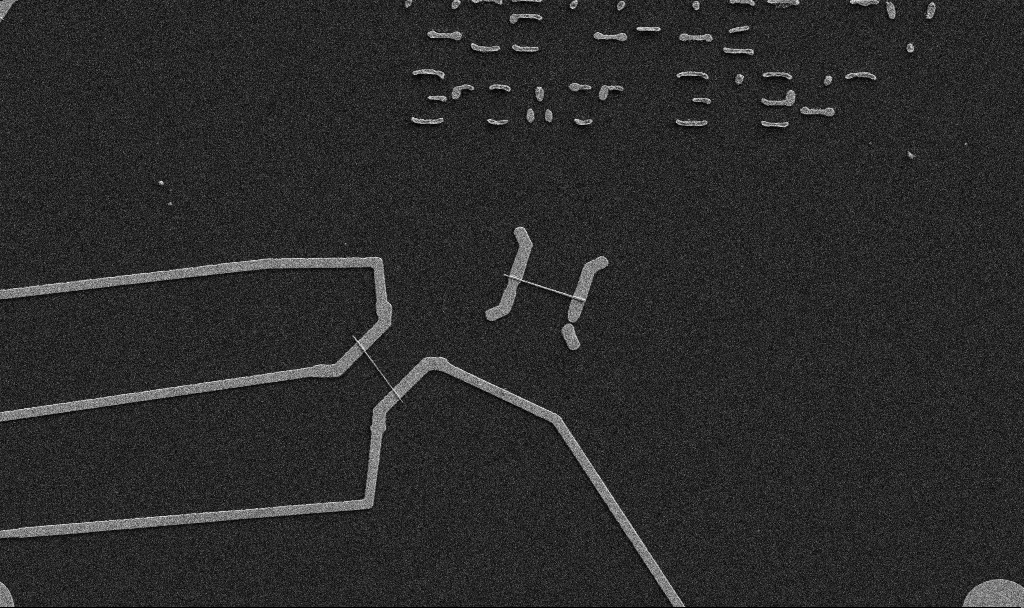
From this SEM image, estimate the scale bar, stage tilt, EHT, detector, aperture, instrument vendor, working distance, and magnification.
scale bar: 10000 nm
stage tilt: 0°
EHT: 5 kV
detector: SE2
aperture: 30 µm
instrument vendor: Zeiss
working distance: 10.7 mm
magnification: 5 K X